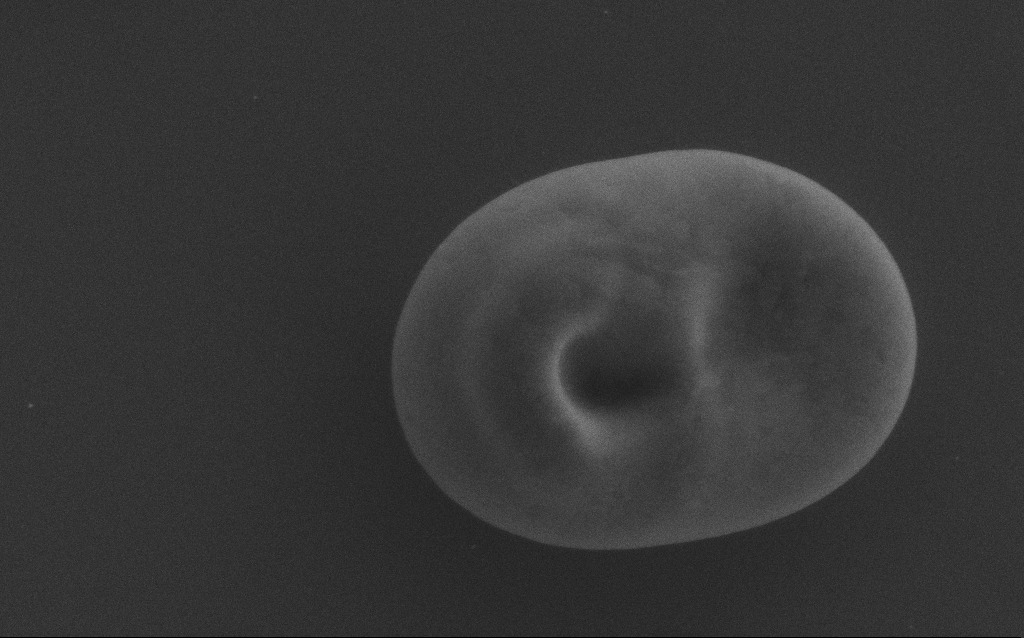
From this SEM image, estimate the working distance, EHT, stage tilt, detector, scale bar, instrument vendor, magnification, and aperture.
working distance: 2 mm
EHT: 5 kV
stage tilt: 0°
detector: SE2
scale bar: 1000 nm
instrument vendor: Zeiss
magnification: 38.07 K X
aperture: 30 µm